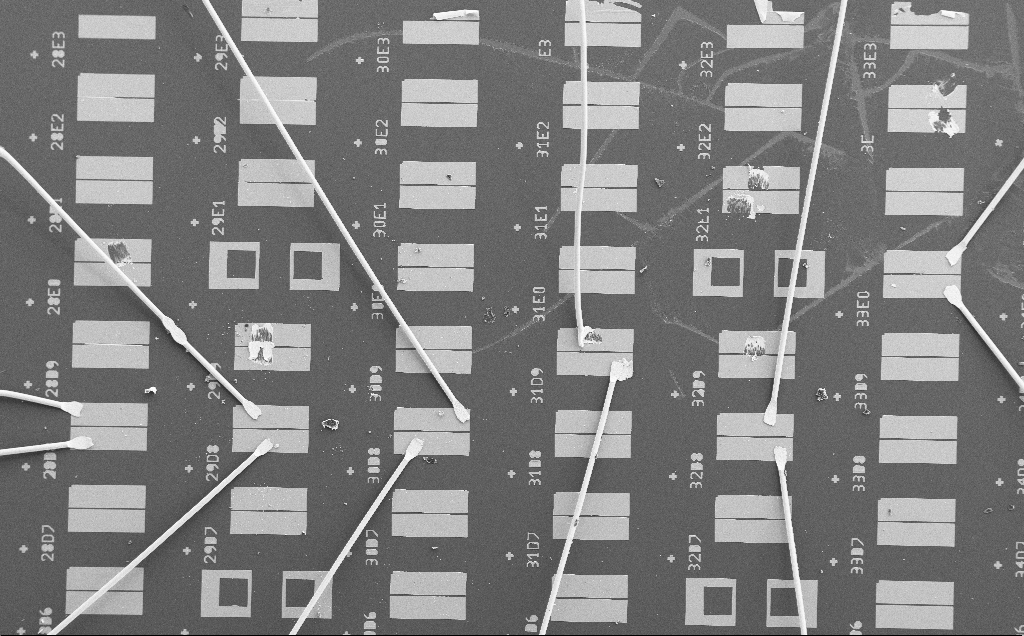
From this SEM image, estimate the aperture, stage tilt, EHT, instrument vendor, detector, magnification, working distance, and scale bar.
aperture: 30 µm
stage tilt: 0°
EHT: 15 kV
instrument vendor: Zeiss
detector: SE2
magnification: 0.087 K X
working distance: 10 mm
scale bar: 200000 nm